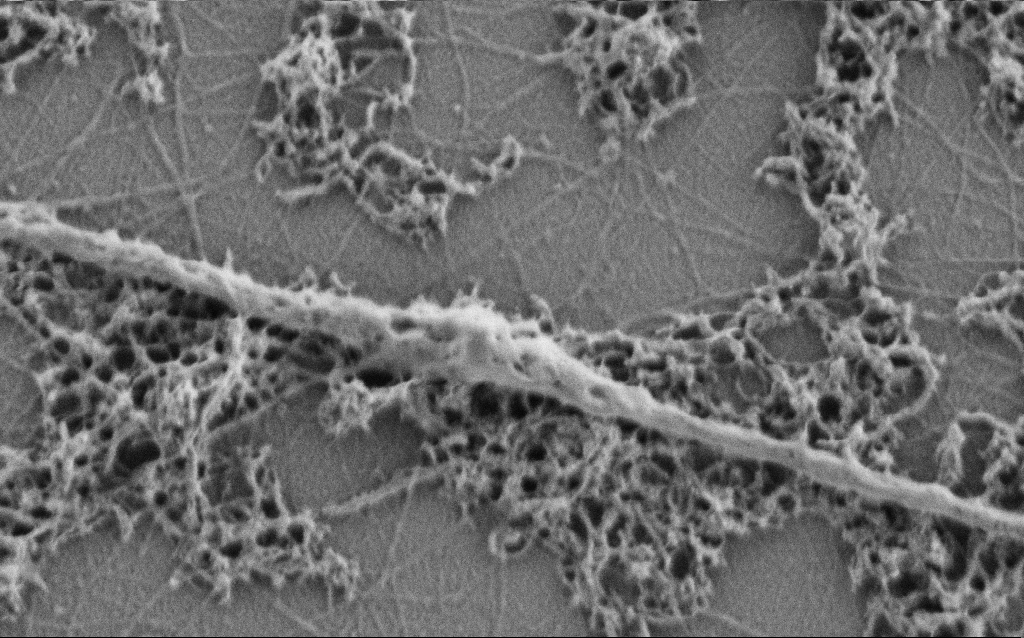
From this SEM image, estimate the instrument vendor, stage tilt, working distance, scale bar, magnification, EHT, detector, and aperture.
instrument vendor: Zeiss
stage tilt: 0°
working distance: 4 mm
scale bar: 1000 nm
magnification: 50 K X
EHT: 0.9 kV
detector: SE2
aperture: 30 µm